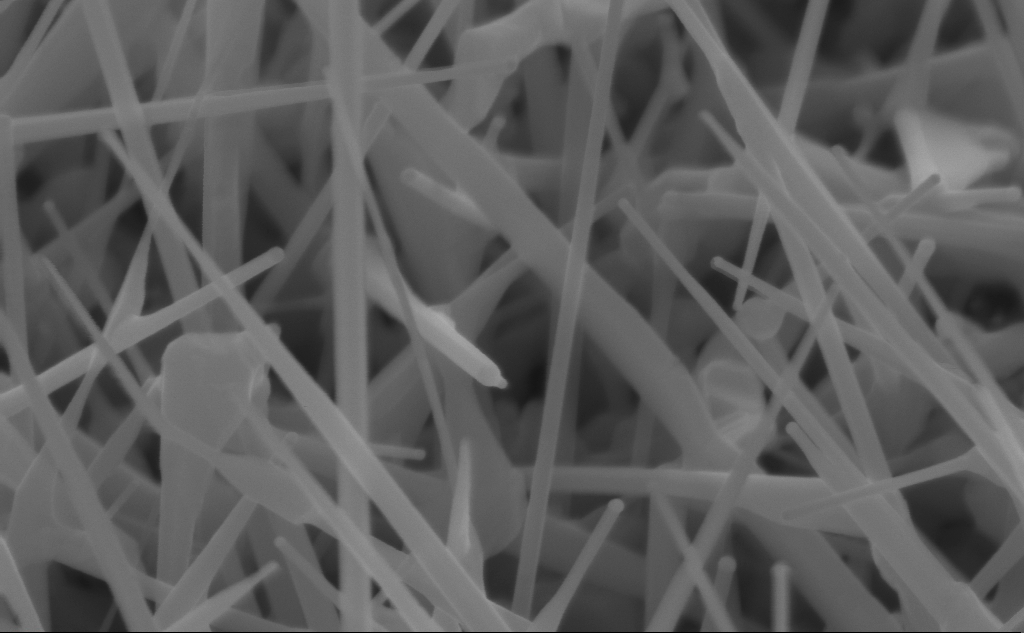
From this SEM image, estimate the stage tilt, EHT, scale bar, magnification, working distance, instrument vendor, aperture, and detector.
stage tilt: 45°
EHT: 10 kV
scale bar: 200 nm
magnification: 150 K X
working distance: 4 mm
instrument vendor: Zeiss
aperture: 30 µm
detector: InLens